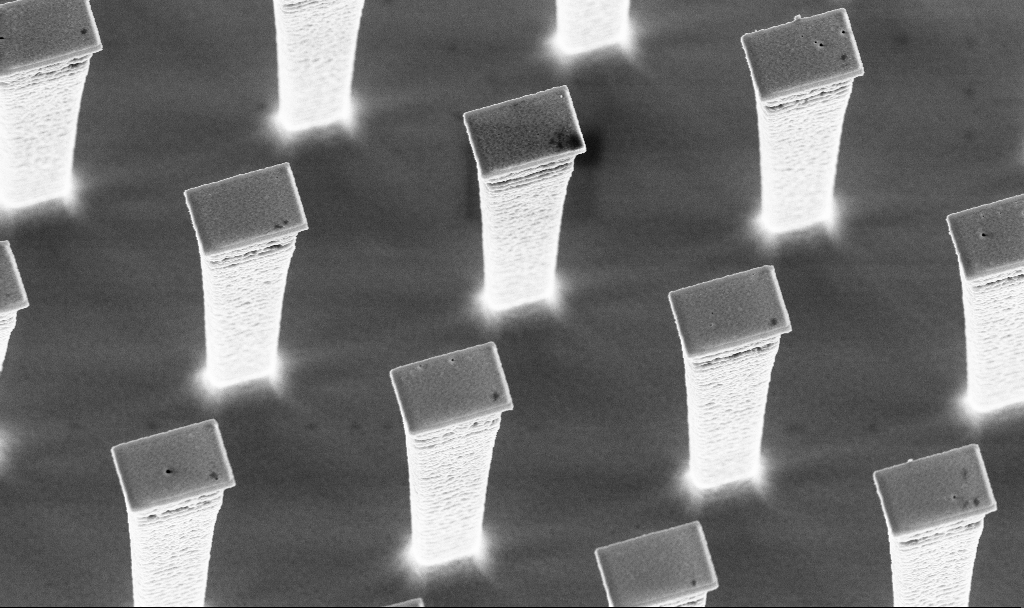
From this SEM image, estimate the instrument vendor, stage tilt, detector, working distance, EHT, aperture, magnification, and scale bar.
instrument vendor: Zeiss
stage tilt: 20°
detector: InLens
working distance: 3 mm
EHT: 5 kV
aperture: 30 µm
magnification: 8.91 K X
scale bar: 2000 nm